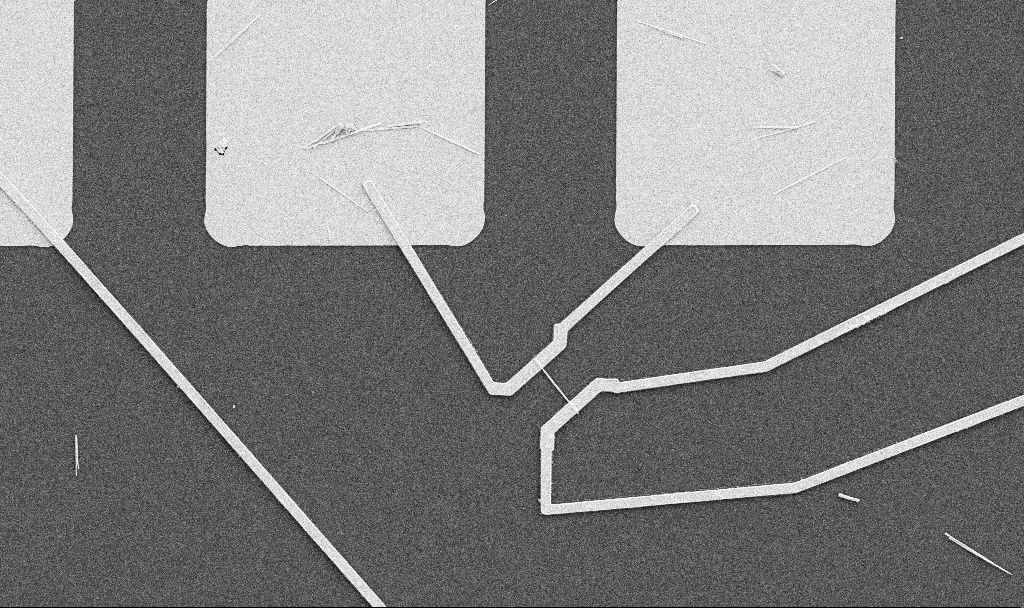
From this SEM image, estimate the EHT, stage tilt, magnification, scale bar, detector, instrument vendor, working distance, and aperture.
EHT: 5 kV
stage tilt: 0°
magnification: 5 K X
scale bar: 10000 nm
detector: SE2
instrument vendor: Zeiss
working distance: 10.7 mm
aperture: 30 µm